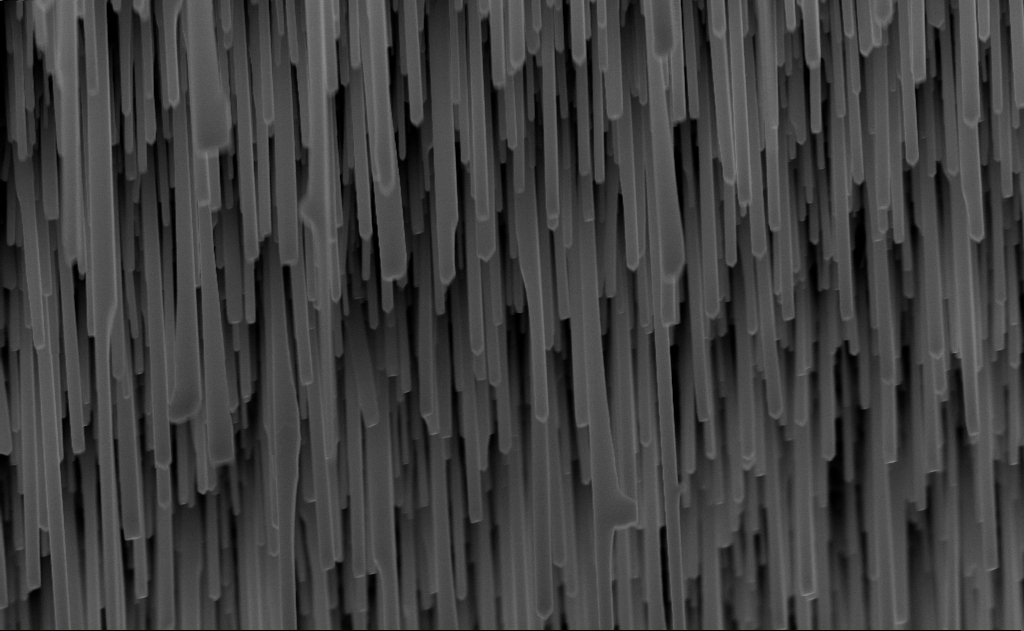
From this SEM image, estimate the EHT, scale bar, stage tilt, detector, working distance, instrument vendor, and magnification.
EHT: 10 kV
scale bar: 1000 nm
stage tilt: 0°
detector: InLens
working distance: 6 mm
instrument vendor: Zeiss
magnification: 40 K X